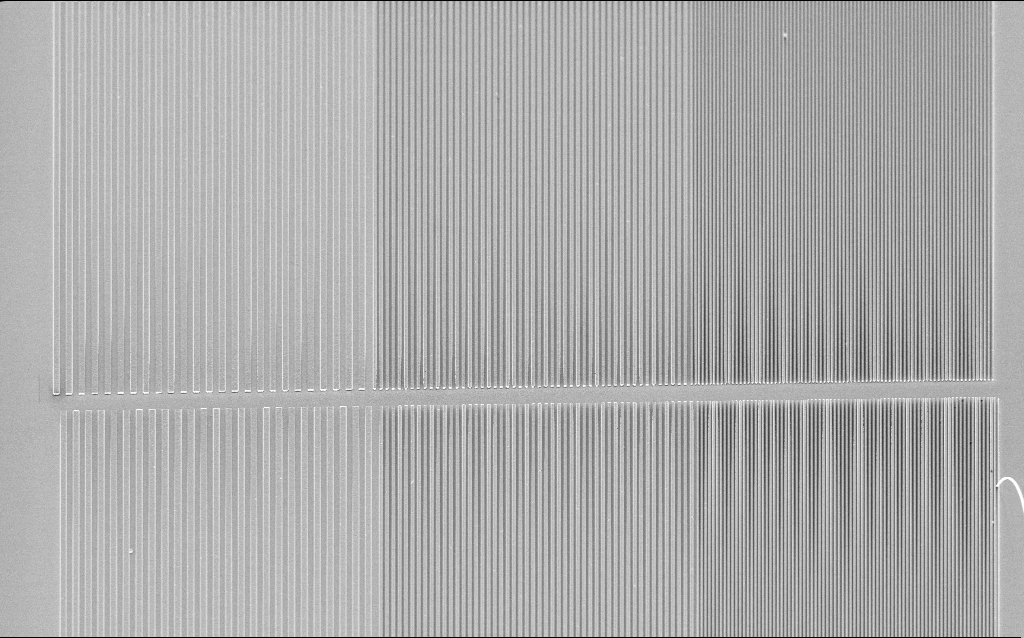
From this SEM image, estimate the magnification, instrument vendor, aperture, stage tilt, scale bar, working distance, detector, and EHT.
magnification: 2.34 K X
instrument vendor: Zeiss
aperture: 30 µm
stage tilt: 30°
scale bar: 10000 nm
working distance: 4.1 mm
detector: InLens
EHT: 5 kV